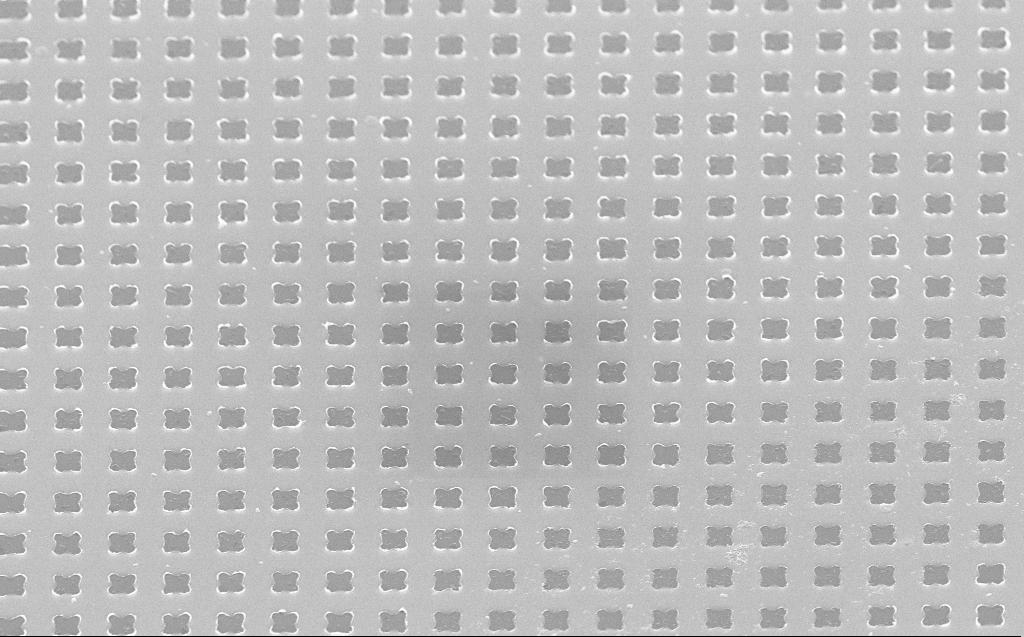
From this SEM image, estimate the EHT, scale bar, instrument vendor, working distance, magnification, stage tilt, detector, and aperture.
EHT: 10 kV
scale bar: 1000 nm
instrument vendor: Zeiss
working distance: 5 mm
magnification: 40.33 K X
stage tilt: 40.6°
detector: InLens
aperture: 30 µm